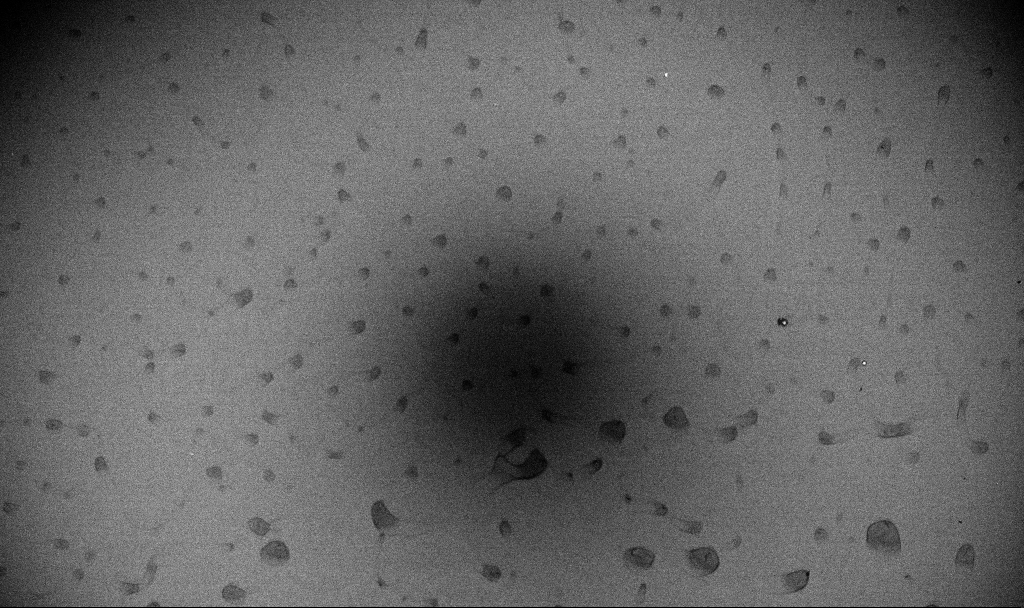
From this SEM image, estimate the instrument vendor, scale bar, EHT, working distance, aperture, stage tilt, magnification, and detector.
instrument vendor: Zeiss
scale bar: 200000 nm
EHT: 10 kV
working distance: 5.8 mm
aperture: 60 µm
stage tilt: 0°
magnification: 0.157 K X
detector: InLens